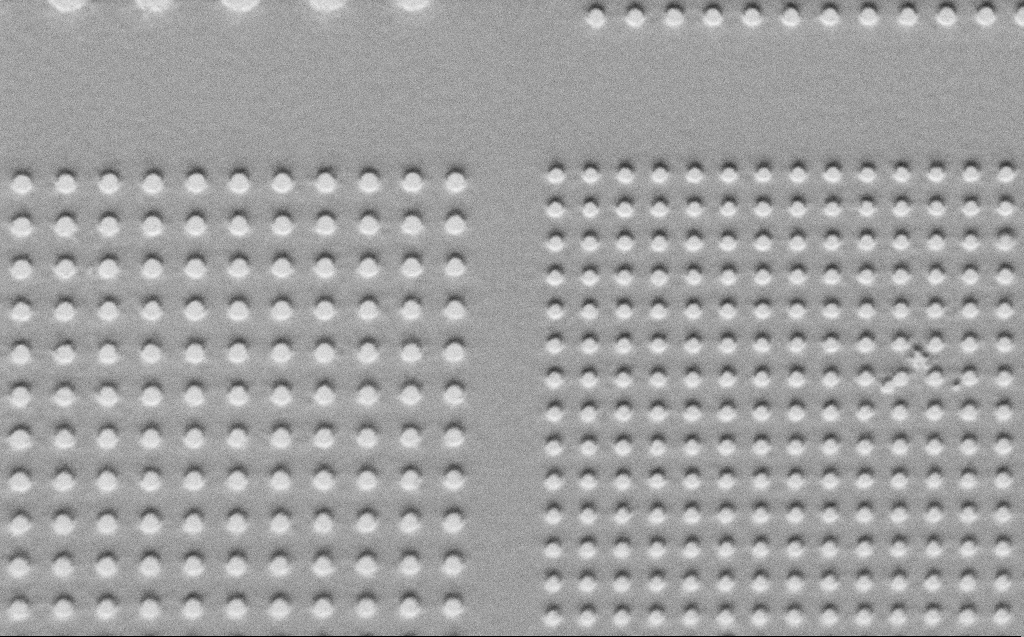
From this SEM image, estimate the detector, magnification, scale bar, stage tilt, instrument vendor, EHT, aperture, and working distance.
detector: SE2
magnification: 8.06 K X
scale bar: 2000 nm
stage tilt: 45°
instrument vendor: Zeiss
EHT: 1 kV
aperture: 30 µm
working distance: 5 mm